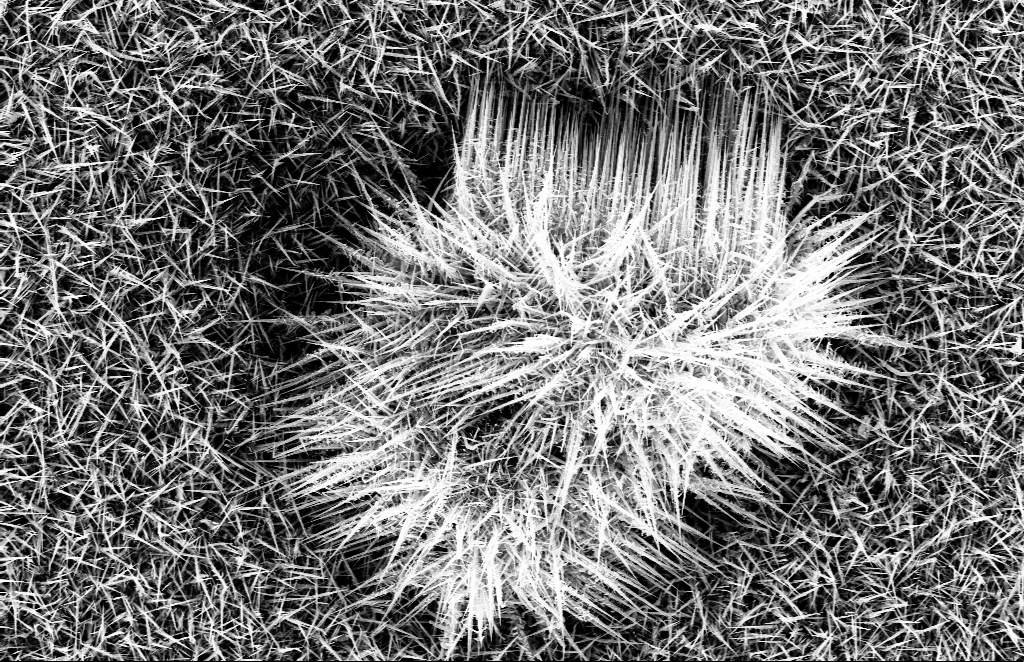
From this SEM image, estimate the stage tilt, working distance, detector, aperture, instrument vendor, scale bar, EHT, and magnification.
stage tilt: -1.1°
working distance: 10 mm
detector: InLens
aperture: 30 µm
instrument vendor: Zeiss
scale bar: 1000 nm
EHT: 10 kV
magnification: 14.54 K X